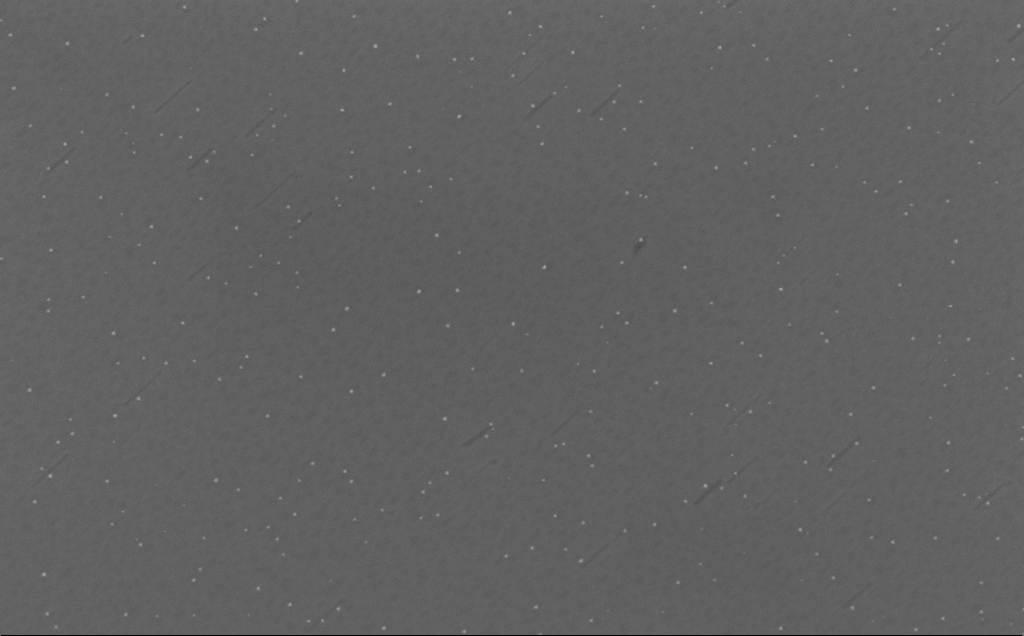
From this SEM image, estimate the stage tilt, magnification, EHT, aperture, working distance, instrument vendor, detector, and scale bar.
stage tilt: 0°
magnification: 157.41 K X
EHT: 10 kV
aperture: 30 µm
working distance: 6 mm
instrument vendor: Zeiss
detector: InLens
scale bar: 100 nm